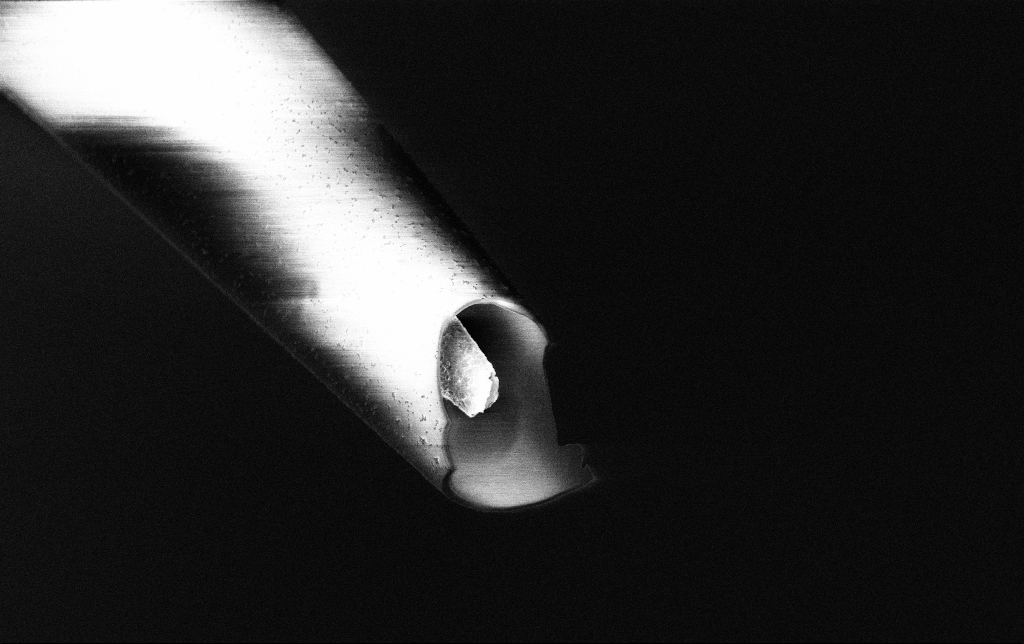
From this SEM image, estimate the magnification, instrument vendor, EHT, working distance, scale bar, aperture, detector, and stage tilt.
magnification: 15 K X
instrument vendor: Zeiss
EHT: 3 kV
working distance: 7.7 mm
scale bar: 2000 nm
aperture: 30 µm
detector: InLens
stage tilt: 45°